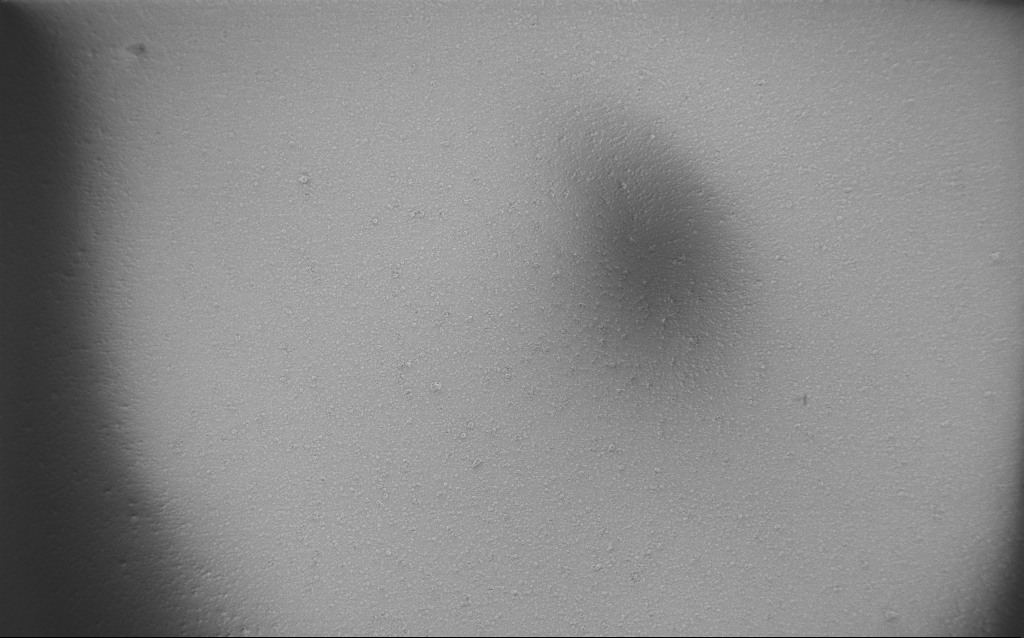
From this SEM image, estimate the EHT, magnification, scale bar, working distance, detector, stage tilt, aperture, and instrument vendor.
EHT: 3 kV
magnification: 0.11 K X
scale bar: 200000 nm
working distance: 2.4 mm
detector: InLens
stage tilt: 0°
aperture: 20 µm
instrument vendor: Zeiss